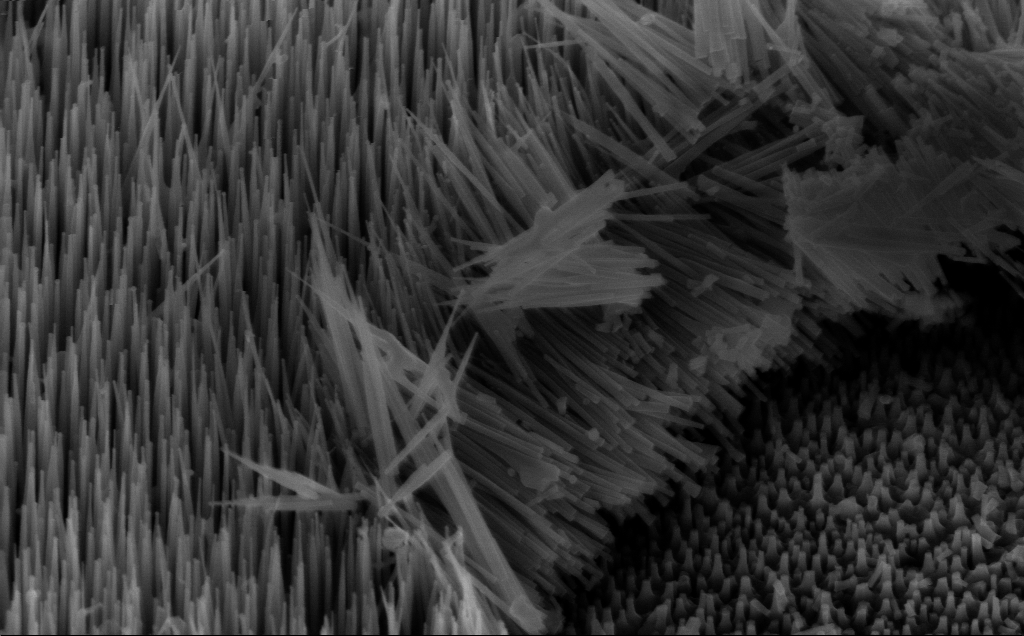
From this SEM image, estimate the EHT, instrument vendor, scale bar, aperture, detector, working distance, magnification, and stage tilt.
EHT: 10 kV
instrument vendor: Zeiss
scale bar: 1000 nm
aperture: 30 µm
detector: InLens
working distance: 5 mm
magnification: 58.84 K X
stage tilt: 45°